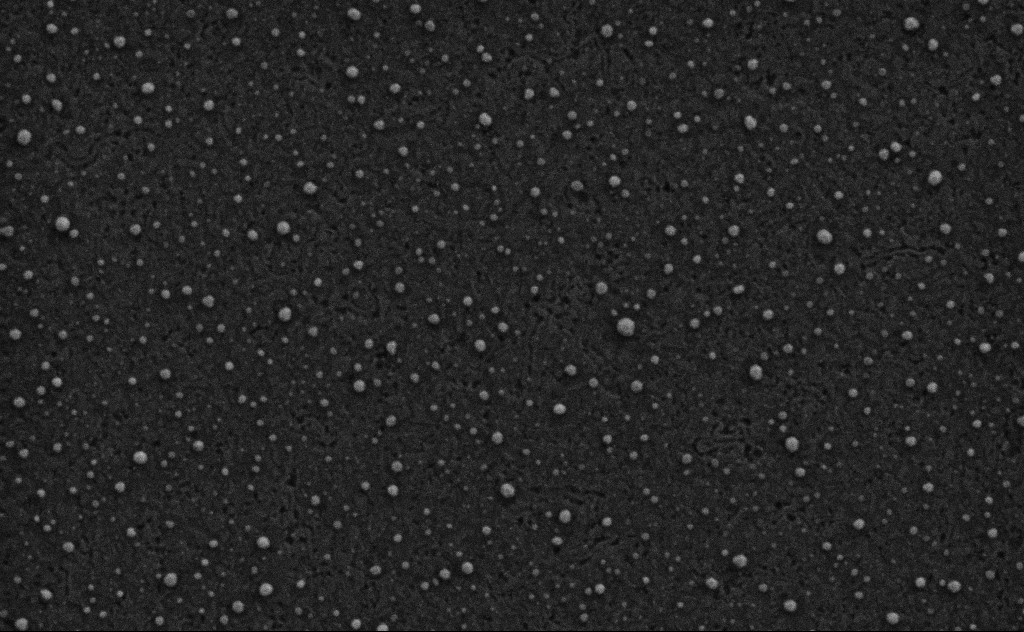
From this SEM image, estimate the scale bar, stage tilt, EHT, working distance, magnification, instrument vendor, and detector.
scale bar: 200 nm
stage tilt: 0°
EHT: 3 kV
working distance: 4 mm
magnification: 80 K X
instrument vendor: Zeiss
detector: SE2